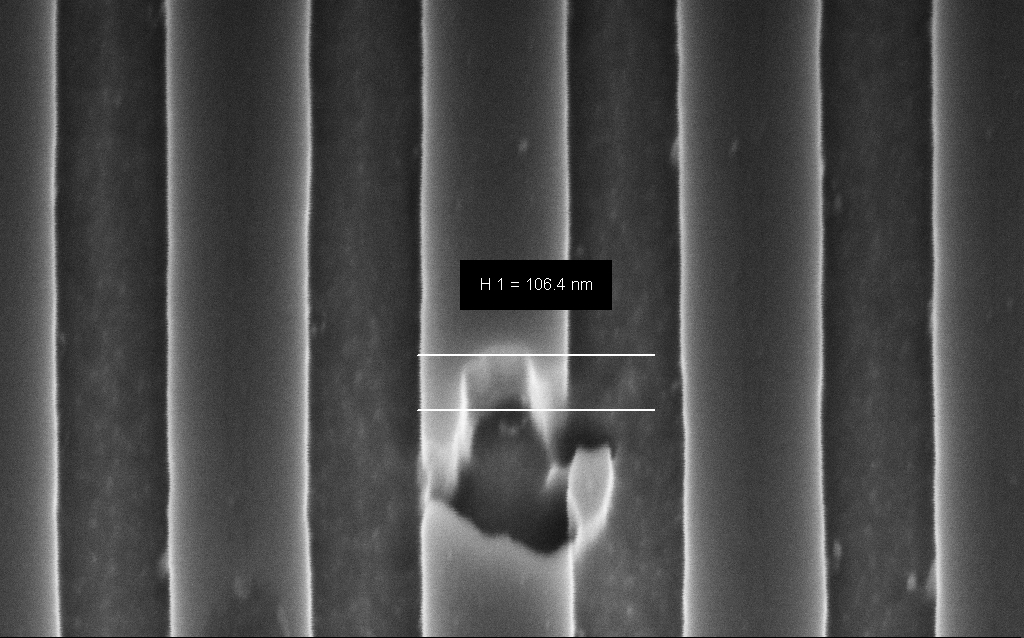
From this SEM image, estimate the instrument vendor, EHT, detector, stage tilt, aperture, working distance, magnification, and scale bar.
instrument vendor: Zeiss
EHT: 3 kV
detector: InLens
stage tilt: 45°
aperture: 30 µm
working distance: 6 mm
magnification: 189.83 K X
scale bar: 200 nm